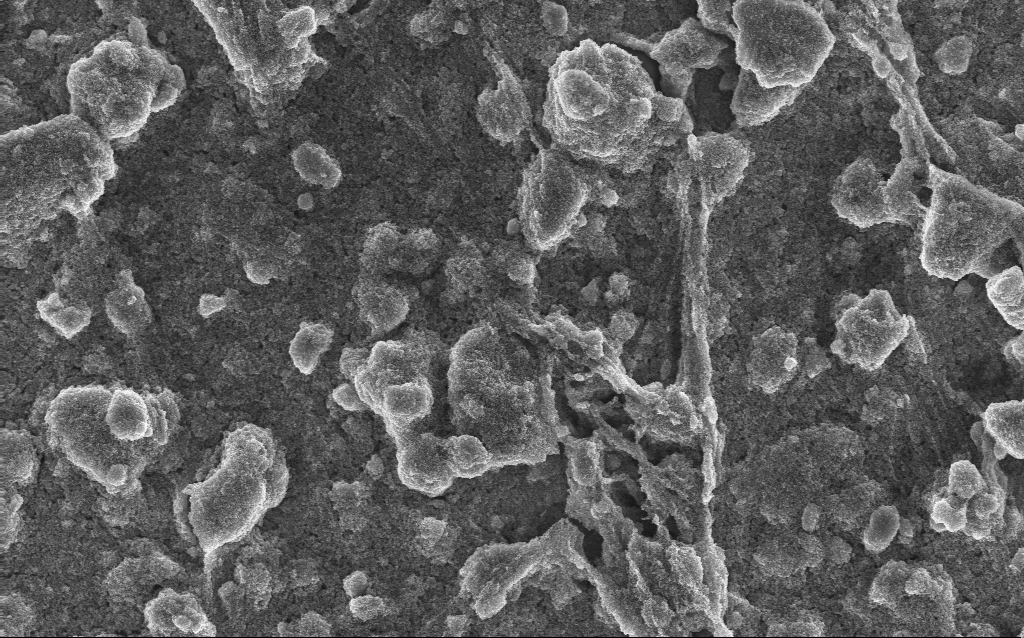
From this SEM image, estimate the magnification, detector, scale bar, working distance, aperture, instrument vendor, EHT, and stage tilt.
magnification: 5.83 K X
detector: InLens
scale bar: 10000 nm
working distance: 2.8 mm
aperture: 30 µm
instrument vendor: Zeiss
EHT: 10 kV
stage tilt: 0°